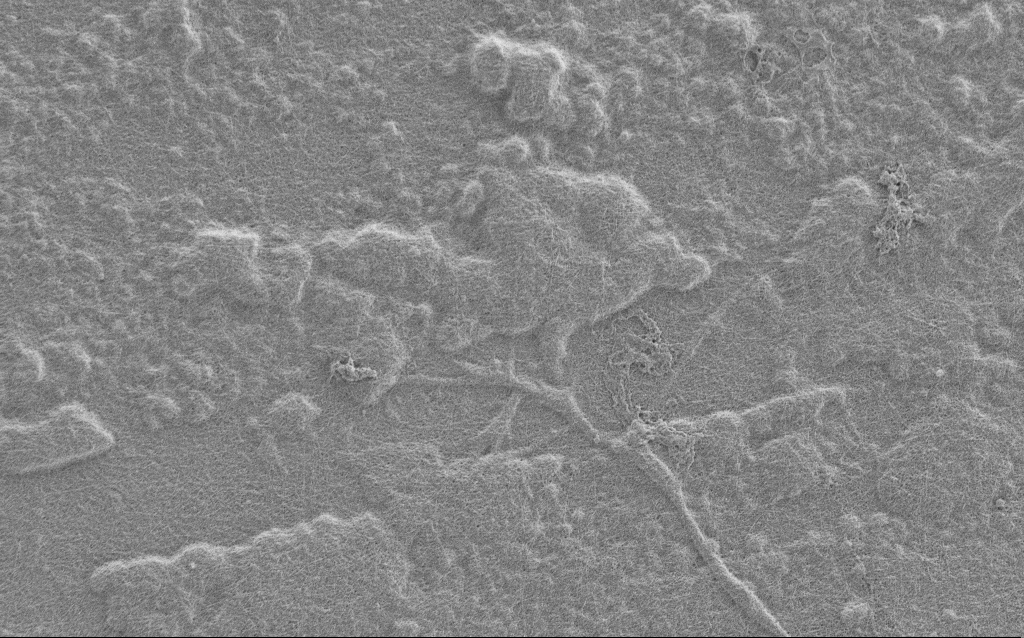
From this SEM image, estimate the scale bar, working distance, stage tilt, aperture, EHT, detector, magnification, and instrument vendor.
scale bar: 2000 nm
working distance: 6 mm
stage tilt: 0°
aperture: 30 µm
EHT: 1 kV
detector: SE2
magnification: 7.5 K X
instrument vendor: Zeiss